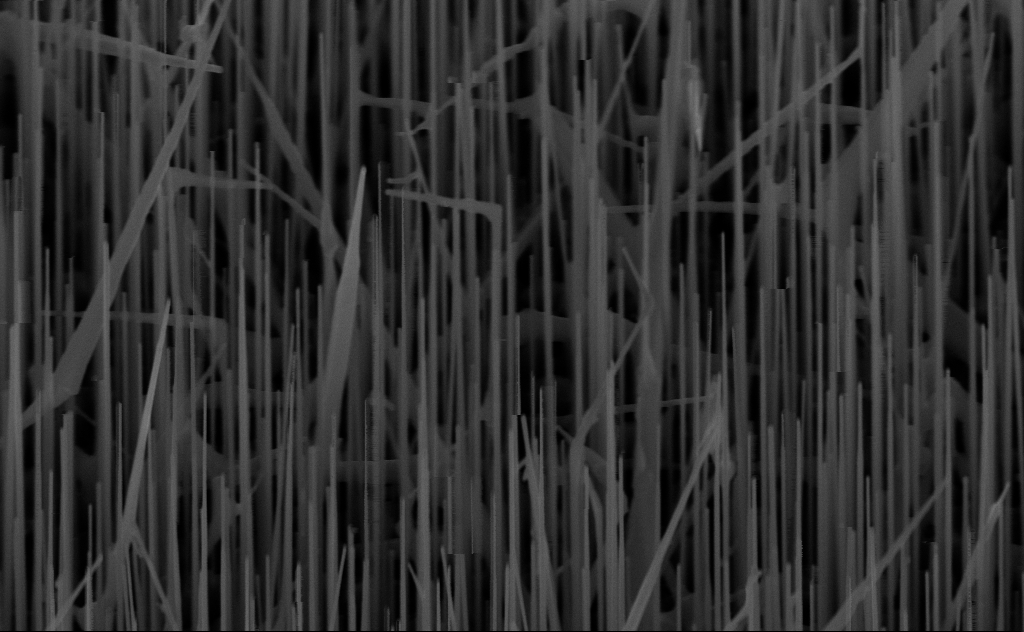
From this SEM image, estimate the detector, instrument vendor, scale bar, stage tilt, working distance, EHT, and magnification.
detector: InLens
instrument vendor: Zeiss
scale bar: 1000 nm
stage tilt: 45°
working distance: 8 mm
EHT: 10 kV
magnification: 40 K X